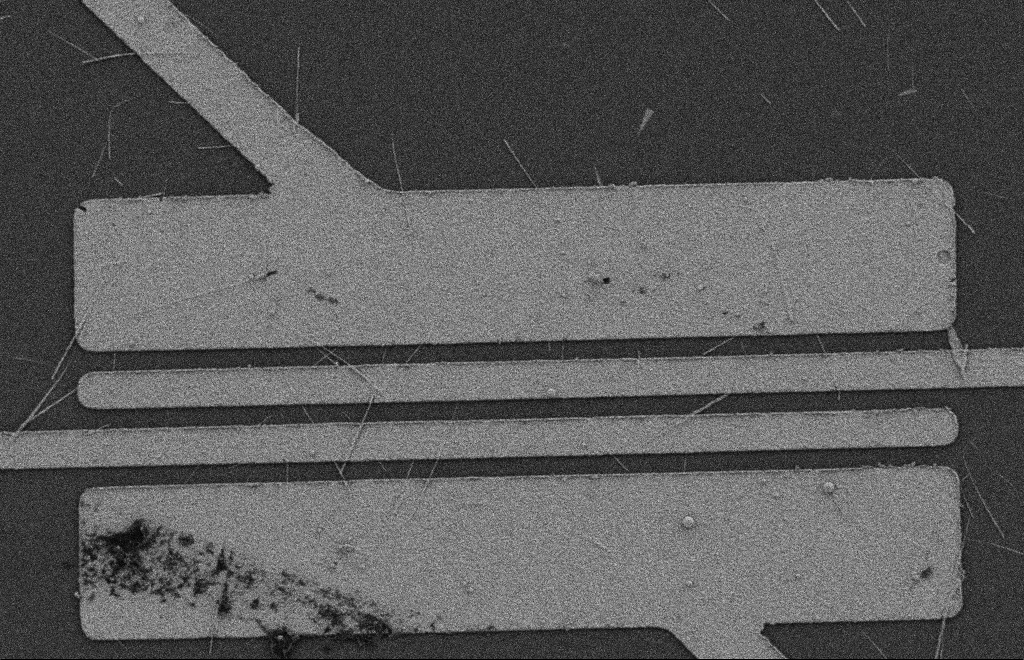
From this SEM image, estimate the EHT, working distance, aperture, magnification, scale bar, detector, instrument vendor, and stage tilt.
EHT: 2 kV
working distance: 9 mm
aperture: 20 µm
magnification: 5.29 K X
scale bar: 2000 nm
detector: SE2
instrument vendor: Zeiss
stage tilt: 0°